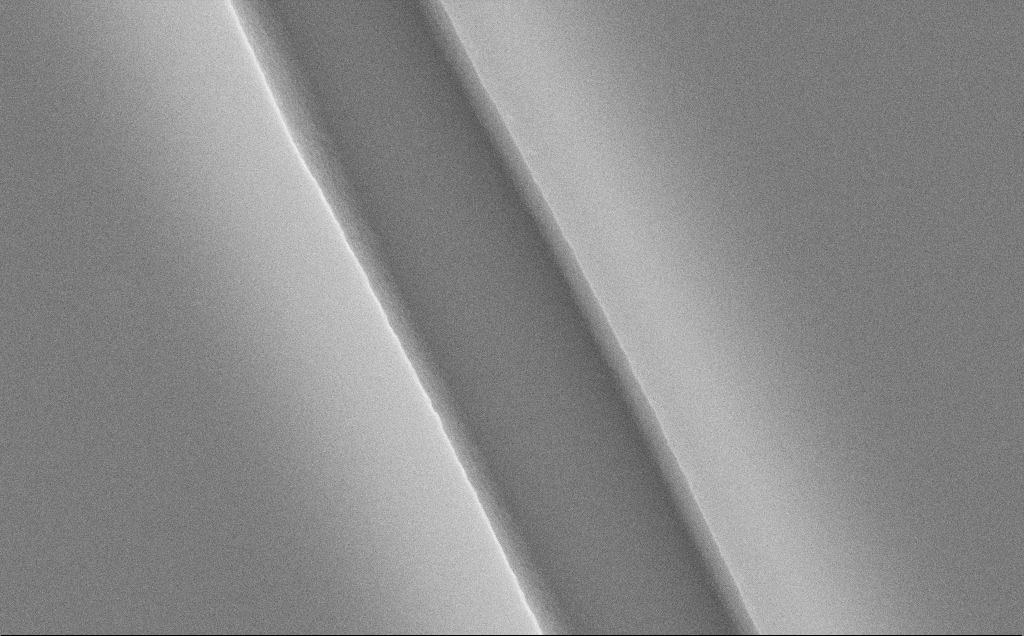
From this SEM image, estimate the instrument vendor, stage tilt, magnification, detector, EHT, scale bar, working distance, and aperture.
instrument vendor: Zeiss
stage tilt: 0°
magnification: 44.6 K X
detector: SE2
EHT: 10 kV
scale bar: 1000 nm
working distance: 12 mm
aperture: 30 µm